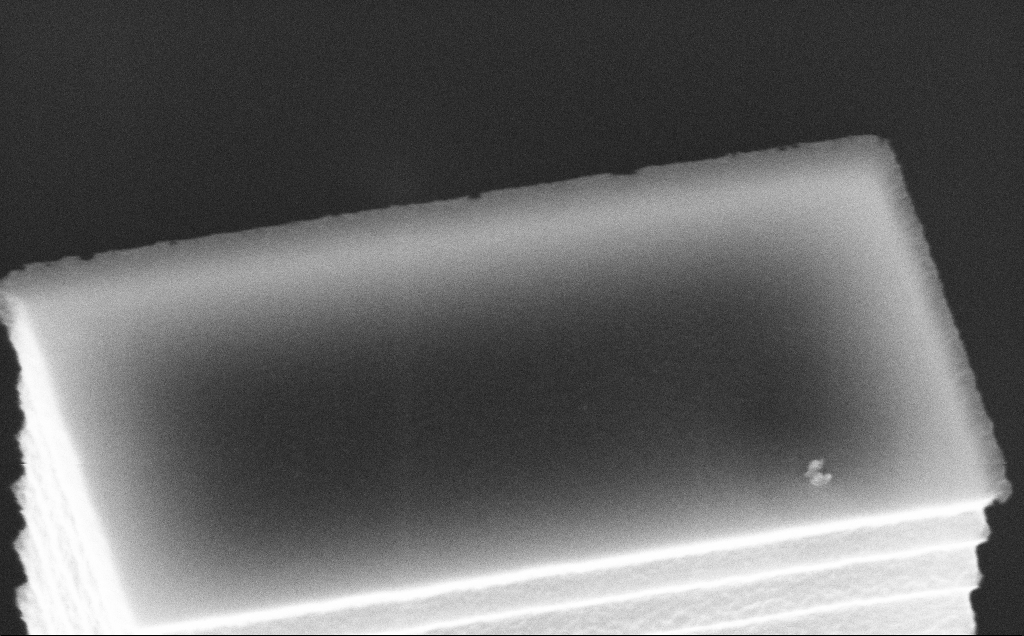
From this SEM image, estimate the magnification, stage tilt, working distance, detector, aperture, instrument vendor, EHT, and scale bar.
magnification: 70.09 K X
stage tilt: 45°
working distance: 7 mm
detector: InLens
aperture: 30 µm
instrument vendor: Zeiss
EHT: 10 kV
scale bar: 1000 nm